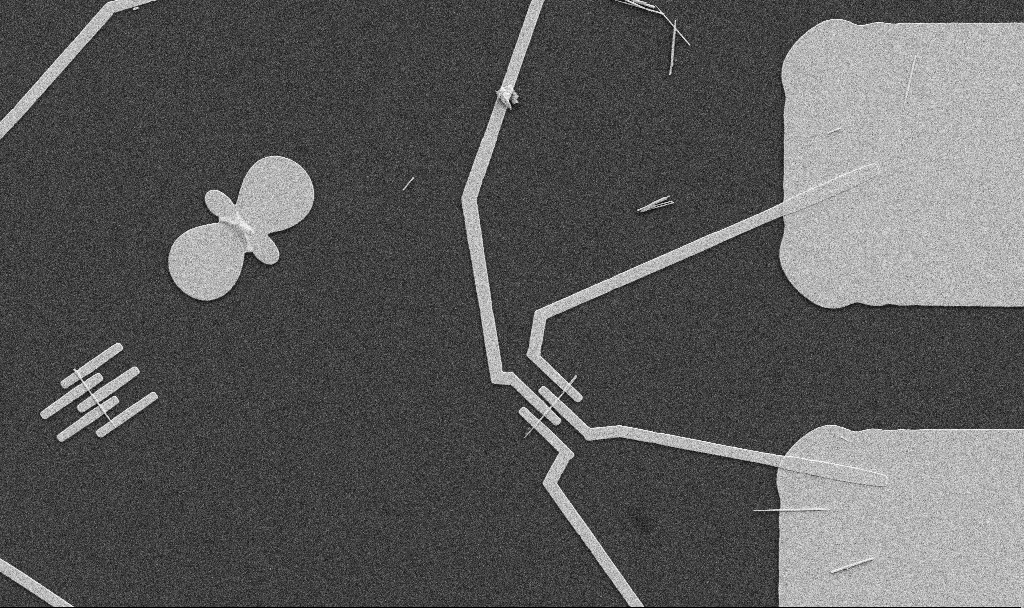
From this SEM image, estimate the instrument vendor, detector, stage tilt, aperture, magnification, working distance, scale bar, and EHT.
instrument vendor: Zeiss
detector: SE2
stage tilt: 0°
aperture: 30 µm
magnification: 5 K X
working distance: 10.7 mm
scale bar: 10000 nm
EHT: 5 kV